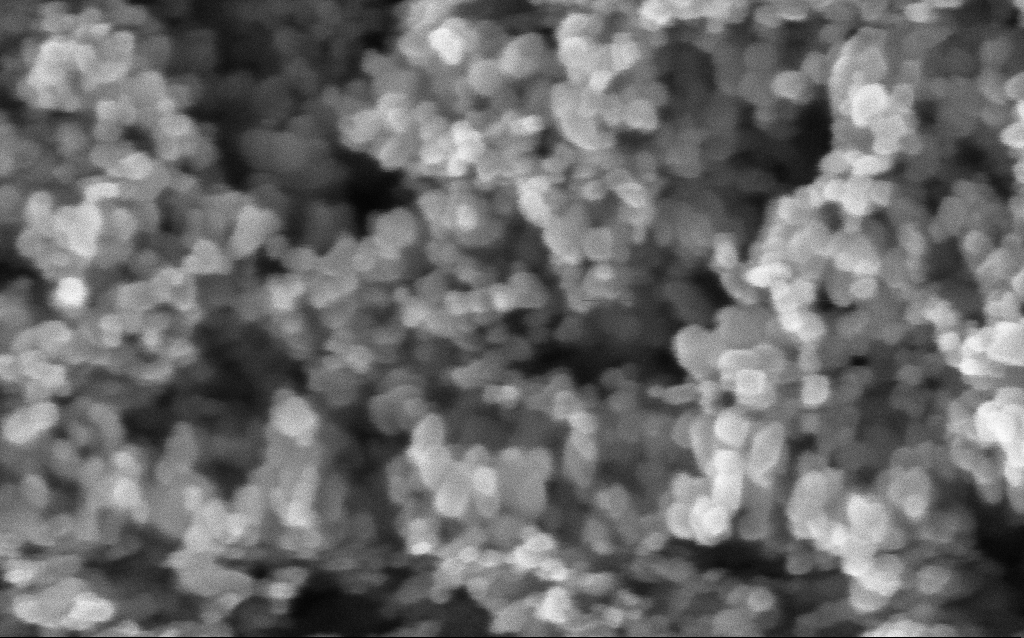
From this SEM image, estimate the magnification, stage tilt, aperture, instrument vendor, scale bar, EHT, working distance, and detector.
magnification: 416 K X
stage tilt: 0°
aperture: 30 µm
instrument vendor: Zeiss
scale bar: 100 nm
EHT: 5 kV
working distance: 2.9 mm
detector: InLens